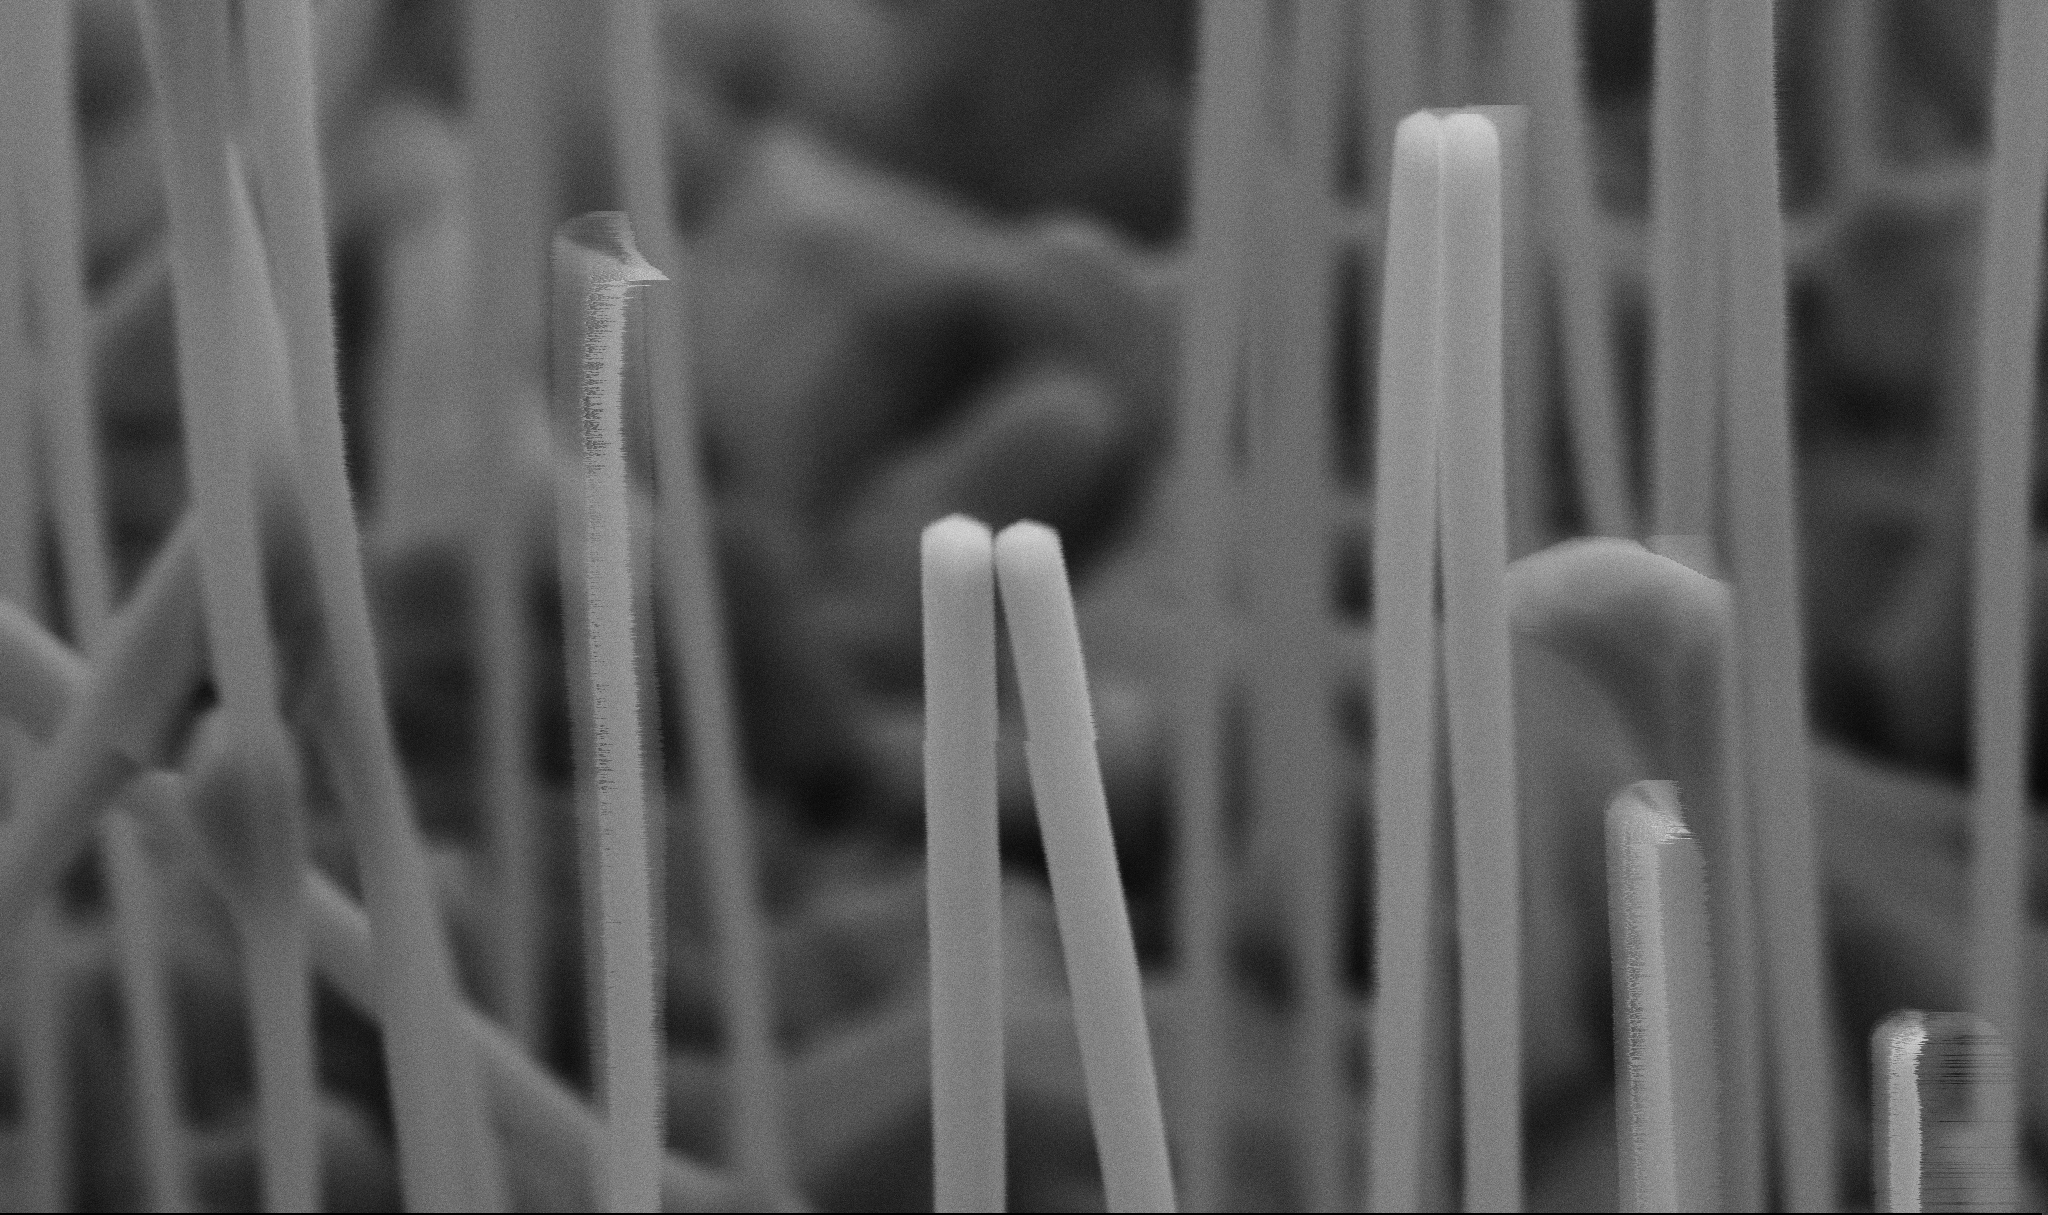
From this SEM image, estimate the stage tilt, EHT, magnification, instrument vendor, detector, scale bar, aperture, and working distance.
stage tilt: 45°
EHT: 5 kV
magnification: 100 K X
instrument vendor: Zeiss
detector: SE2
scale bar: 200 nm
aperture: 30 µm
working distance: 7.3 mm